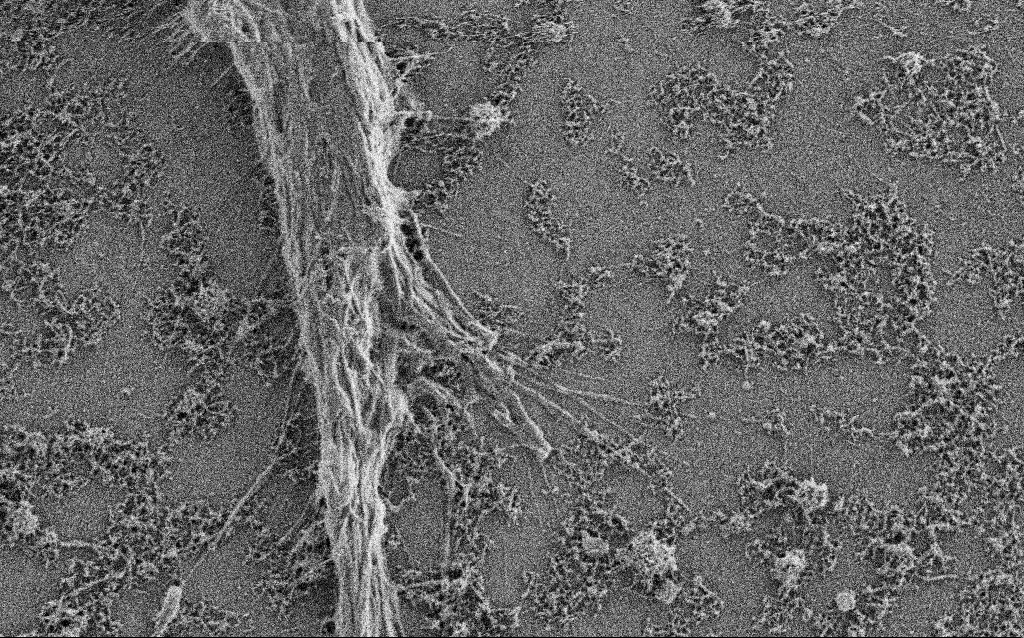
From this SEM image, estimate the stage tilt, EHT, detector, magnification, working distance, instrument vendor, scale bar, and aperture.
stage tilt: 0°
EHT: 0.9 kV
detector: SE2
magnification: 10 K X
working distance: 4 mm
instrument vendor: Zeiss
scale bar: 2000 nm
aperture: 30 µm